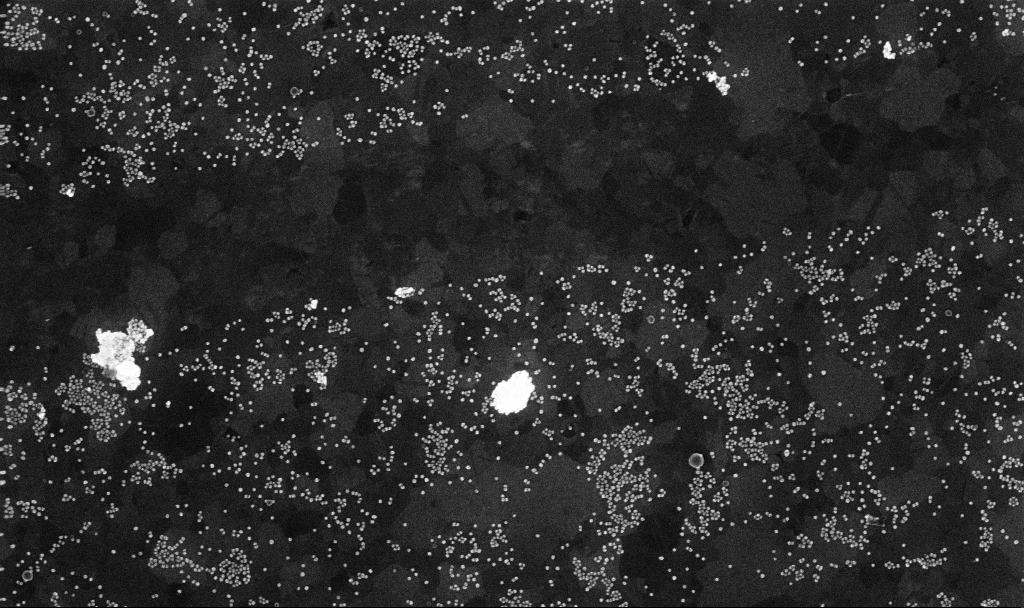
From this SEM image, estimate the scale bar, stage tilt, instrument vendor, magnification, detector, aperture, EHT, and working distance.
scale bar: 1000 nm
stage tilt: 0°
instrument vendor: Zeiss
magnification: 70 K X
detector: InLens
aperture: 30 µm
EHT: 10 kV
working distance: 3.7 mm